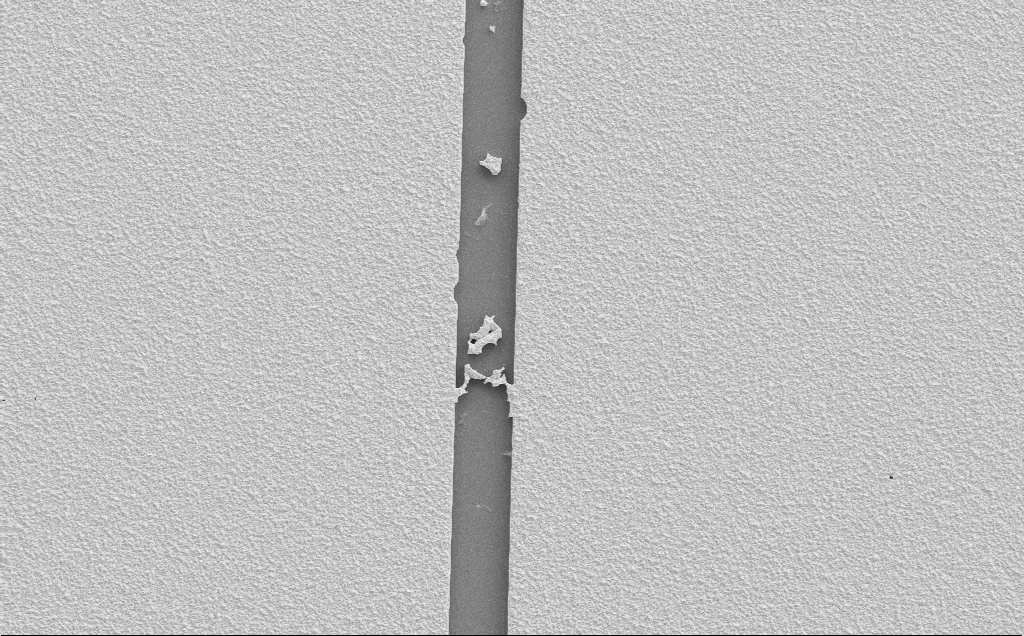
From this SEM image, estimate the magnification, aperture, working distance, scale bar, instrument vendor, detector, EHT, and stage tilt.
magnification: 2.1 K X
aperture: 30 µm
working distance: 9 mm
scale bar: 20000 nm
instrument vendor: Zeiss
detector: SE2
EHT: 10 kV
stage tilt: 0°